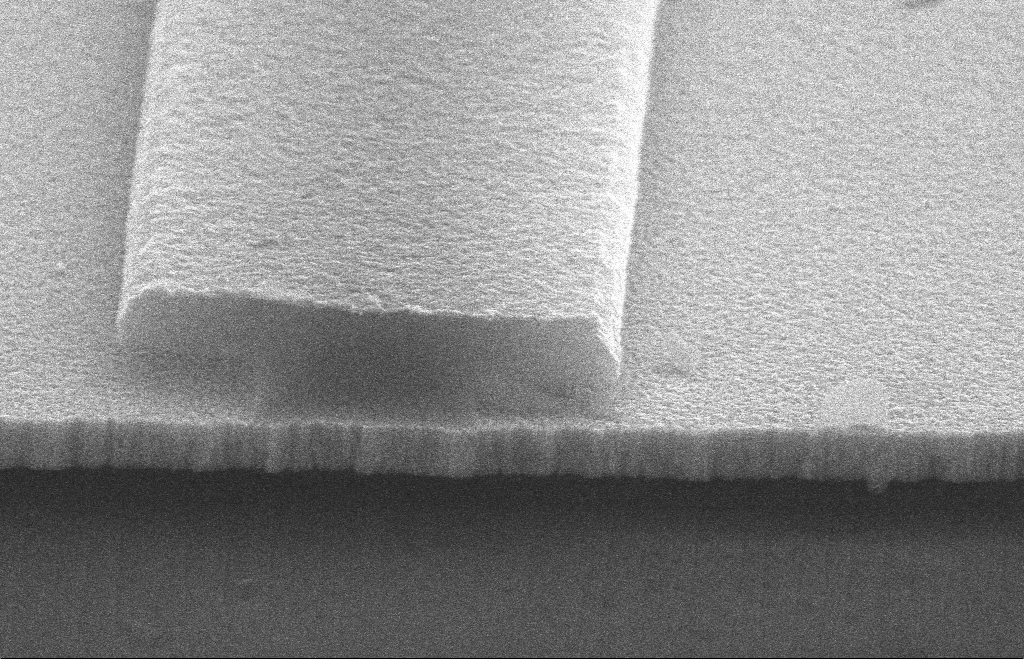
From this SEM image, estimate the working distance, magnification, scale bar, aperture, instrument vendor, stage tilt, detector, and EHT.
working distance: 12 mm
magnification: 49.35 K X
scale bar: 200 nm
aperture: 30 µm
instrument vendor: Zeiss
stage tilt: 65°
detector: SE2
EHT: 10 kV